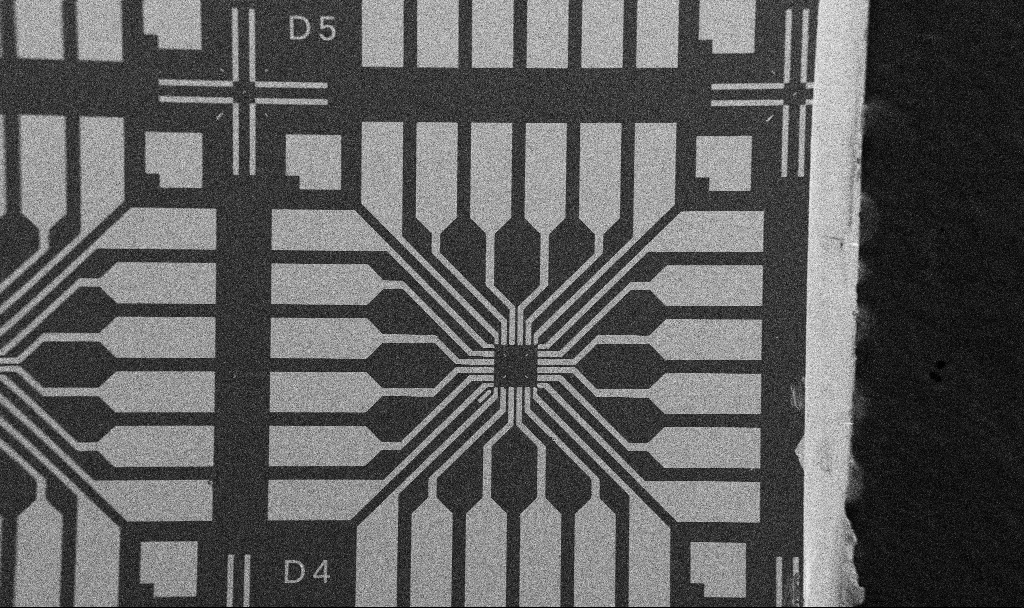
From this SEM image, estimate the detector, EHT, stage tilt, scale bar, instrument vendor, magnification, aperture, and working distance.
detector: SE2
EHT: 5 kV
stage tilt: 0°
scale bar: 200000 nm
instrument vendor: Zeiss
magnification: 0.1 K X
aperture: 30 µm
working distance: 10.7 mm